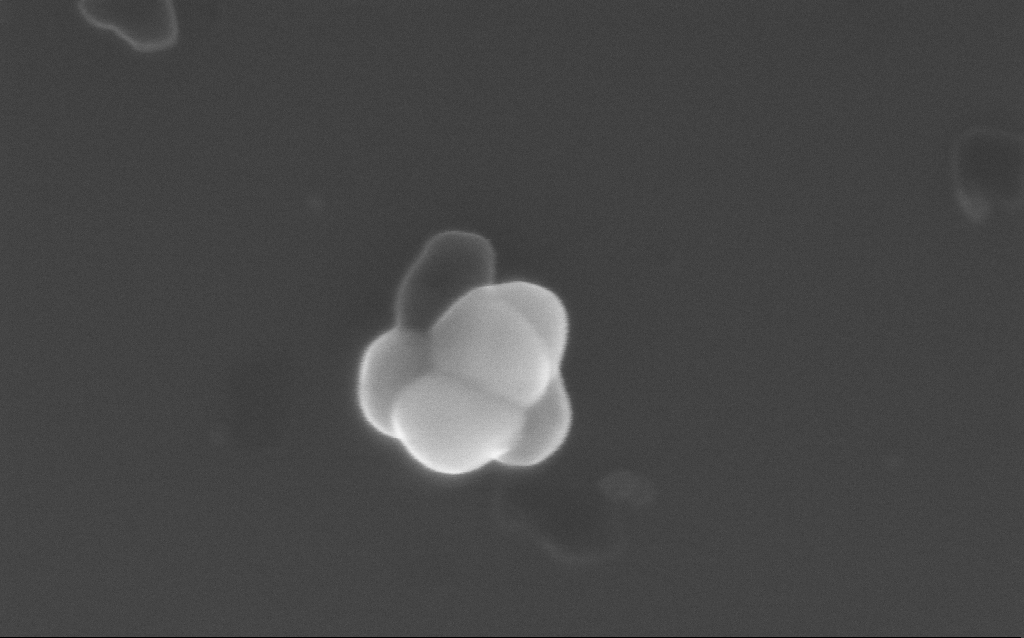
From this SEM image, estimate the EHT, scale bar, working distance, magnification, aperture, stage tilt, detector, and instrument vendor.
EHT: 10 kV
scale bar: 200 nm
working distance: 3 mm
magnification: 222.63 K X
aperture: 30 µm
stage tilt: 0°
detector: InLens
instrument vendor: Zeiss